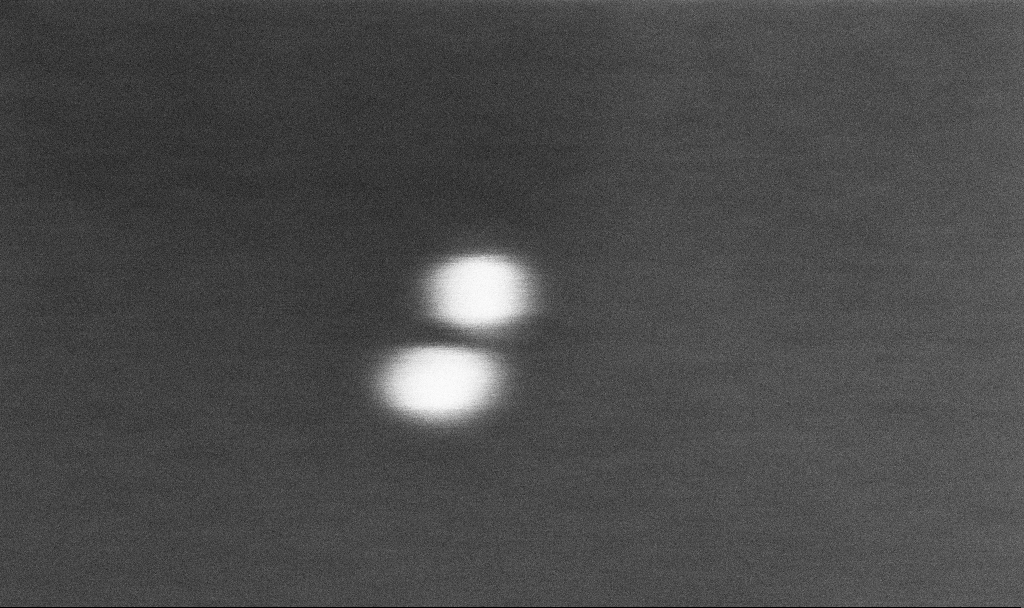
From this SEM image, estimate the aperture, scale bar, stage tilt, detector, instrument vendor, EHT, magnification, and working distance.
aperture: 30 µm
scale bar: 10 nm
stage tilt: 0°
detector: InLens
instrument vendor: Zeiss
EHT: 10 kV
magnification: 1500 K X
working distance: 3.3 mm